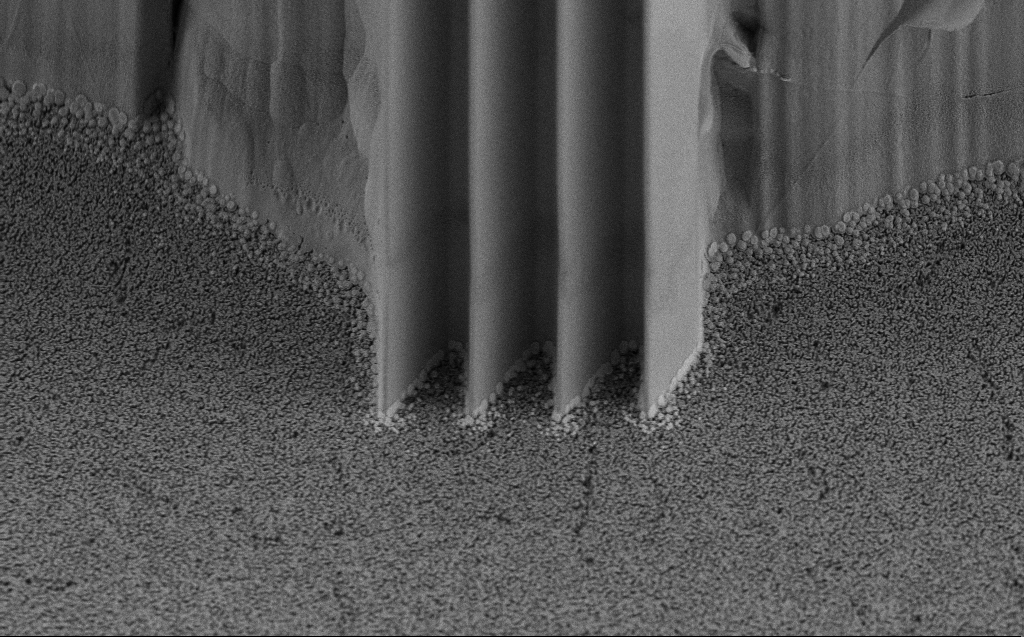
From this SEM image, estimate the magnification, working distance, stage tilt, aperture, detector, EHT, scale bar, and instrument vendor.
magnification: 2.81 K X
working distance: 5 mm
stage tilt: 45°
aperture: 30 µm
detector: SE2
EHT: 5 kV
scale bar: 20000 nm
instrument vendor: Zeiss